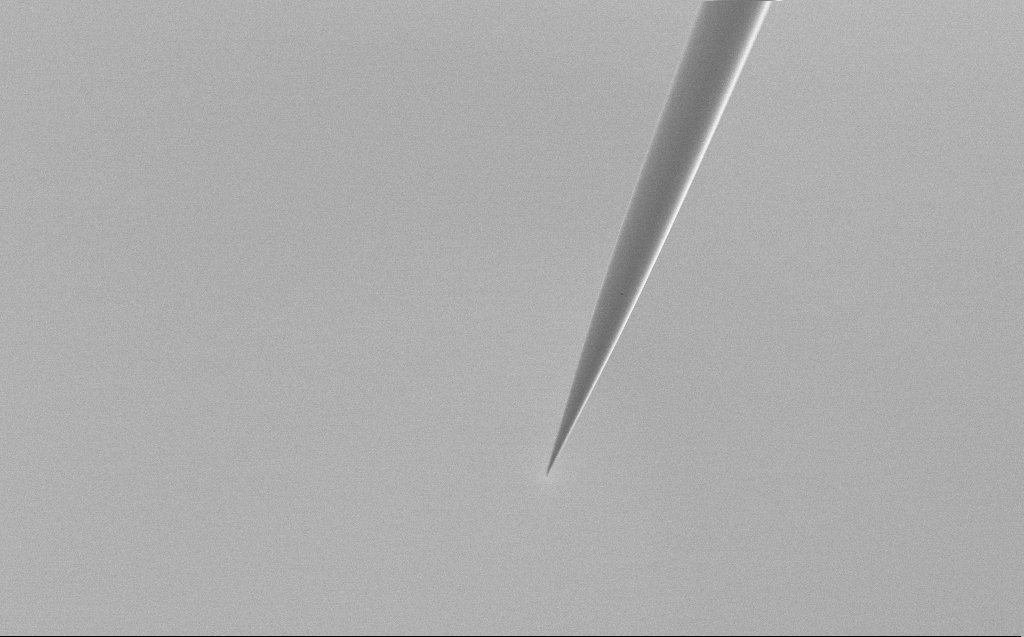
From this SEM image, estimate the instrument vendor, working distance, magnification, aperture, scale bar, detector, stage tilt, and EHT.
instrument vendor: Zeiss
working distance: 5 mm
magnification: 1 K X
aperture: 30 µm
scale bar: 20000 nm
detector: SE2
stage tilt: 45°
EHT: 2 kV